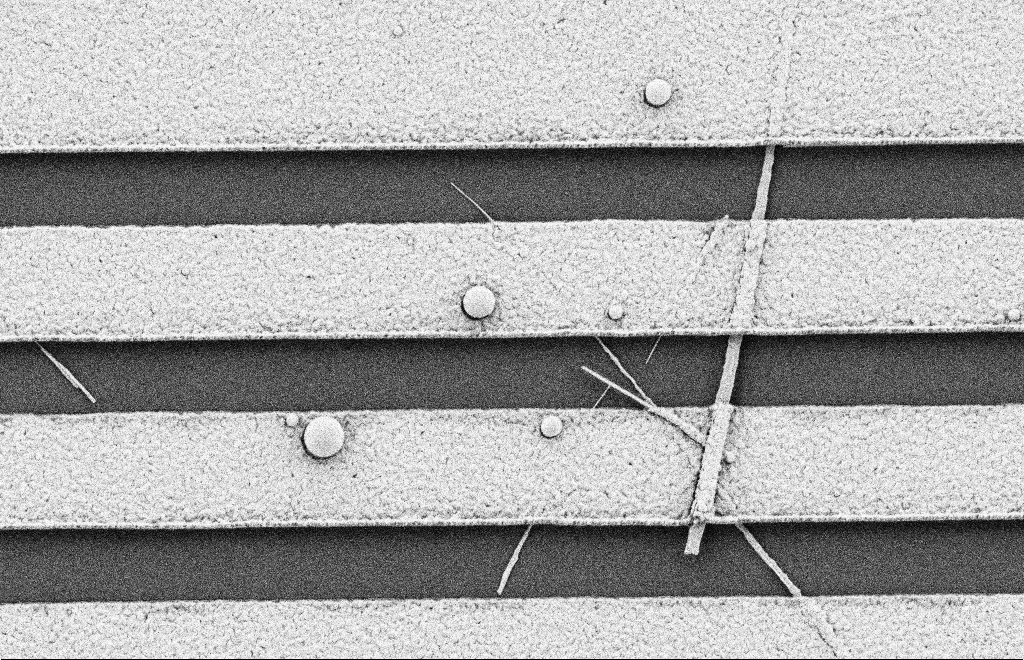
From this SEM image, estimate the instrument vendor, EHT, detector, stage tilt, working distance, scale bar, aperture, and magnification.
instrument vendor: Zeiss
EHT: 2 kV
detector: SE2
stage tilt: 0°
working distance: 10 mm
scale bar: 2000 nm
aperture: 20 µm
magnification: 17.23 K X